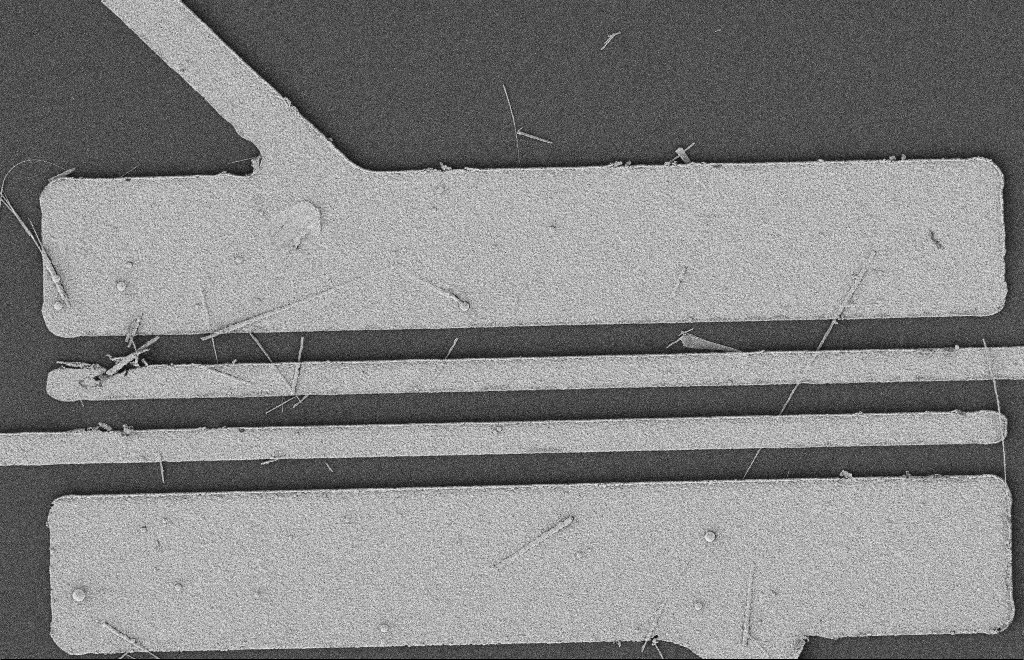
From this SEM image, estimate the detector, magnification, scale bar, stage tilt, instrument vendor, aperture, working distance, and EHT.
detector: SE2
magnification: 5.77 K X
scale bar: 2000 nm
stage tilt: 0°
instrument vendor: Zeiss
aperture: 20 µm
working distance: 12 mm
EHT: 2 kV